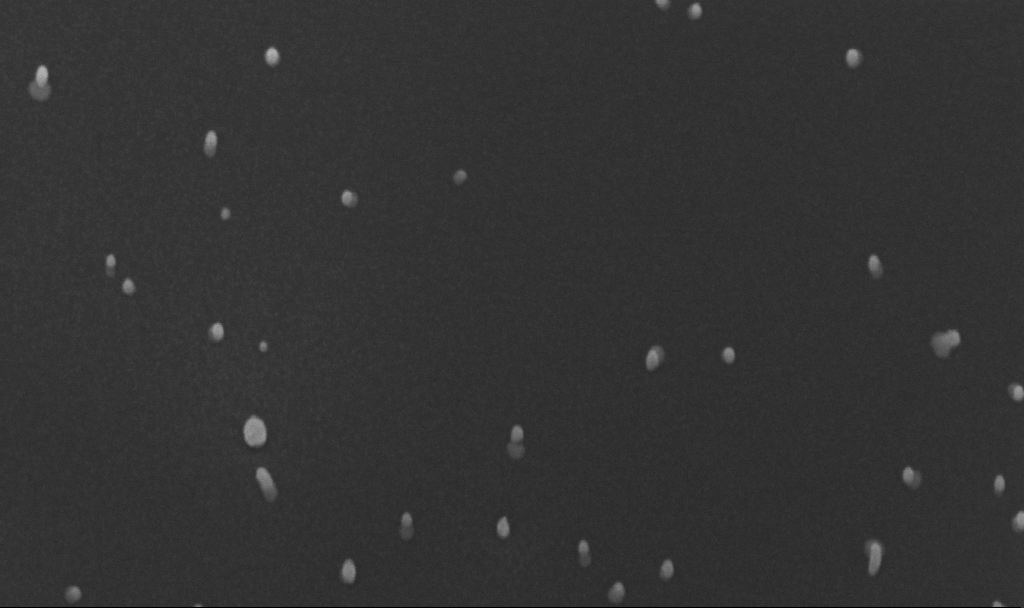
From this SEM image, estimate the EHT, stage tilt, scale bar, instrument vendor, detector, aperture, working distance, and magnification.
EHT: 10 kV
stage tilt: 45°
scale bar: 200 nm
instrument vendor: Zeiss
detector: InLens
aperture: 30 µm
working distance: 4.9 mm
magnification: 200 K X